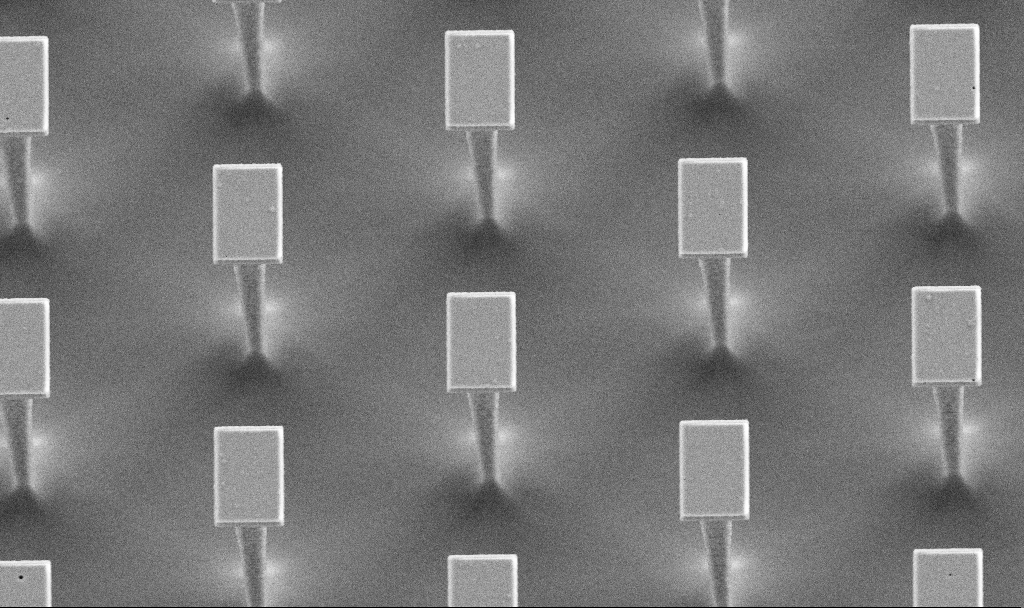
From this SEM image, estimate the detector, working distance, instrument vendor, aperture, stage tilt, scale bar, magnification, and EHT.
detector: SE2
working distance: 4.2 mm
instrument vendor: Zeiss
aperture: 30 µm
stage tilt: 20°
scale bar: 2000 nm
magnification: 8.62 K X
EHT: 5 kV